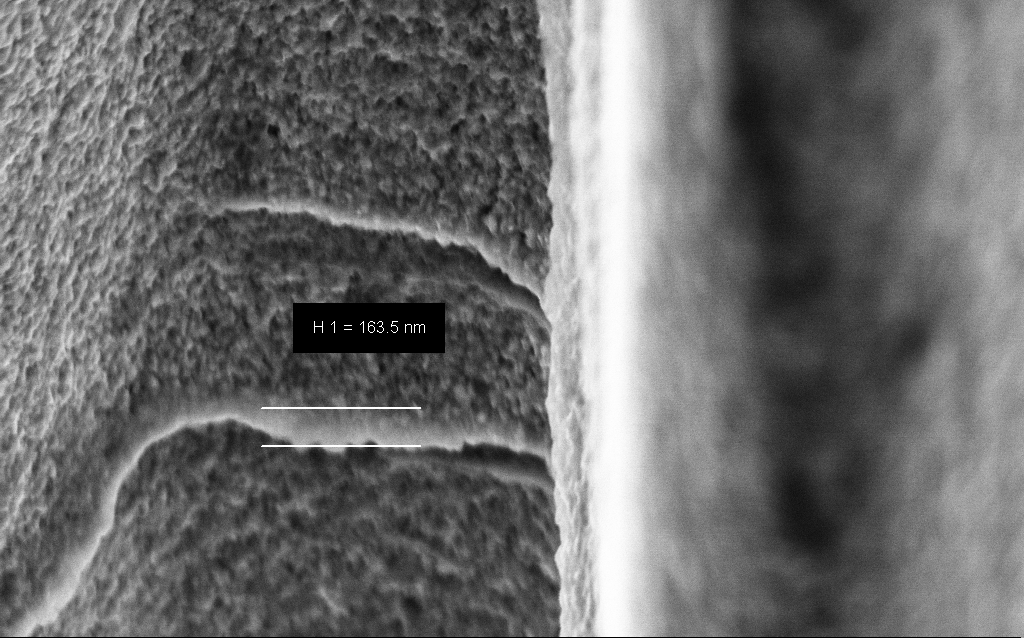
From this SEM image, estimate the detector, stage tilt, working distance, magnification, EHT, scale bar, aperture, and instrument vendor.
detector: InLens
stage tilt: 45°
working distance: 7 mm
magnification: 85.33 K X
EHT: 5 kV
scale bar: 200 nm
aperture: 30 µm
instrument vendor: Zeiss